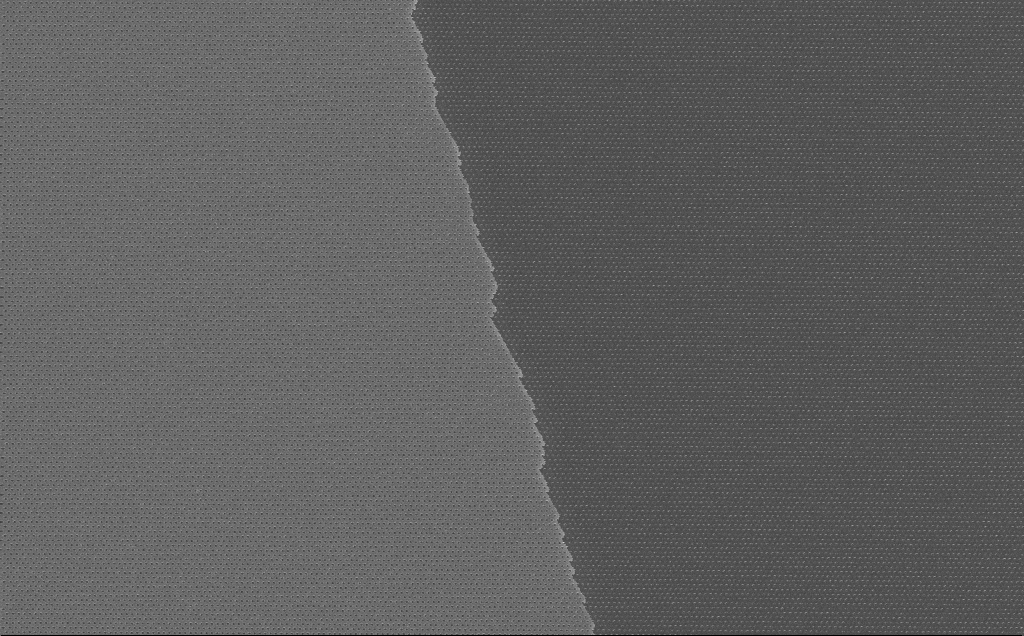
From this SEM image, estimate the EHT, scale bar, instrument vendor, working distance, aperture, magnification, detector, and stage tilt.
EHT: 10 kV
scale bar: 10000 nm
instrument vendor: Zeiss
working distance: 7 mm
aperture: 30 µm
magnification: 6.55 K X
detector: InLens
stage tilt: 0°